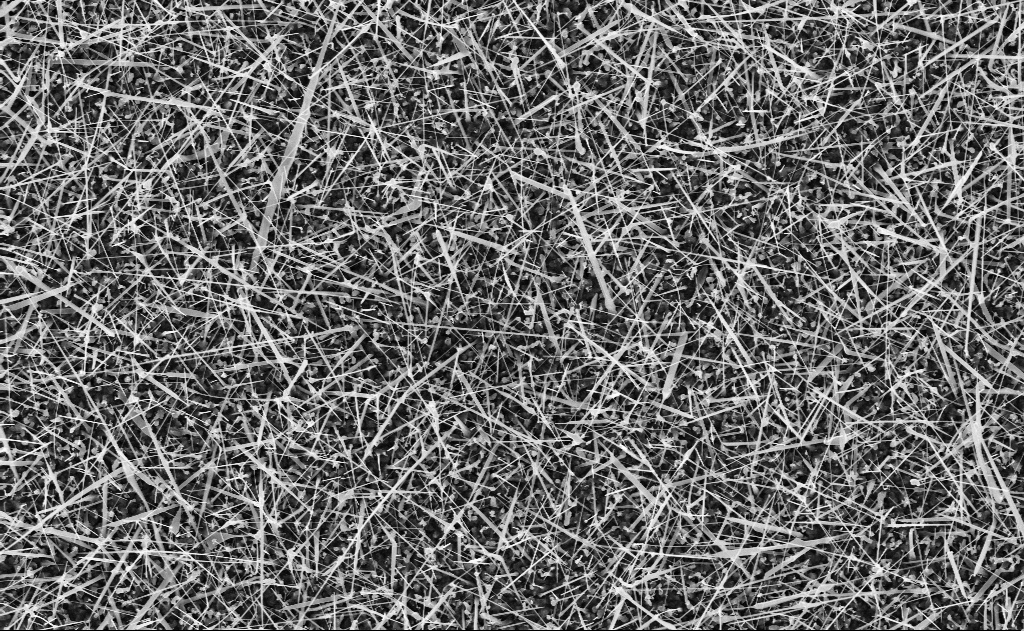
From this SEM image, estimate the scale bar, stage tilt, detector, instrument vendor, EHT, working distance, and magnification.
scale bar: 2000 nm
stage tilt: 0°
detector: InLens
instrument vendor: Zeiss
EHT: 10 kV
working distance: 11 mm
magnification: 10 K X